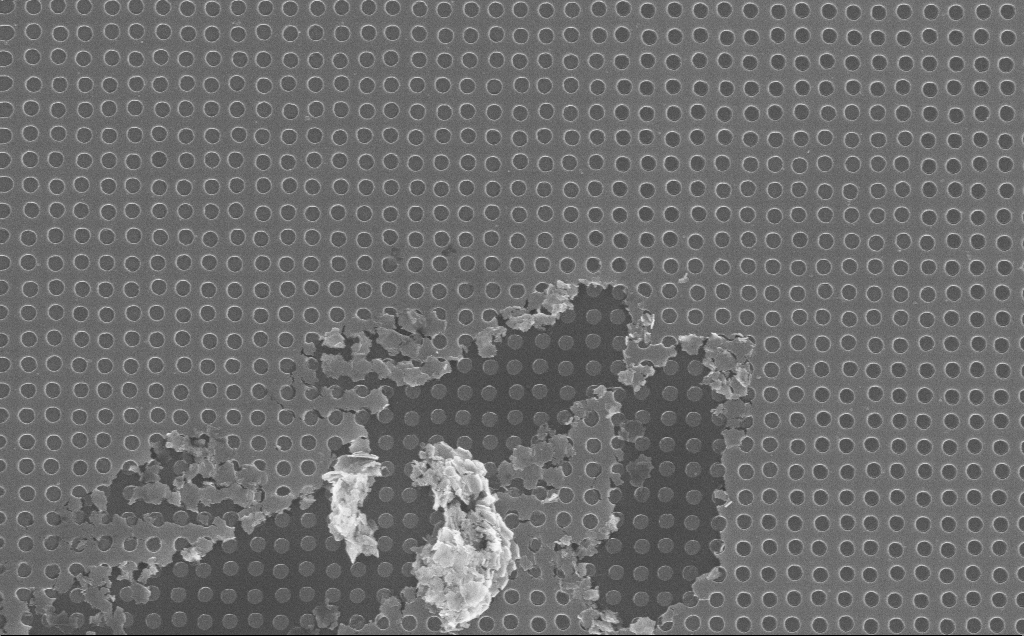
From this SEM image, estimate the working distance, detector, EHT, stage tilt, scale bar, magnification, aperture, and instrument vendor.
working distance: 7 mm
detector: InLens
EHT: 10 kV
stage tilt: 0°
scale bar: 2000 nm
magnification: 23.61 K X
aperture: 30 µm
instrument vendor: Zeiss